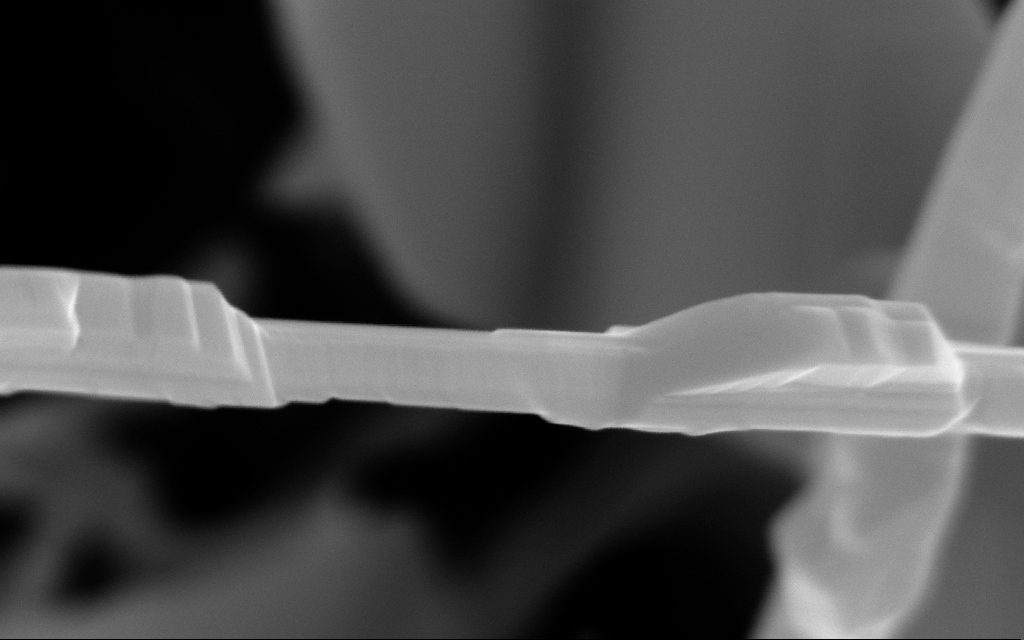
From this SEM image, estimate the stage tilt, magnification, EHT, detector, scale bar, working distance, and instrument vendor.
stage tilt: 0°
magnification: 248.13 K X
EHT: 10 kV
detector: InLens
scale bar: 100 nm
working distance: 6 mm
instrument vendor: Zeiss